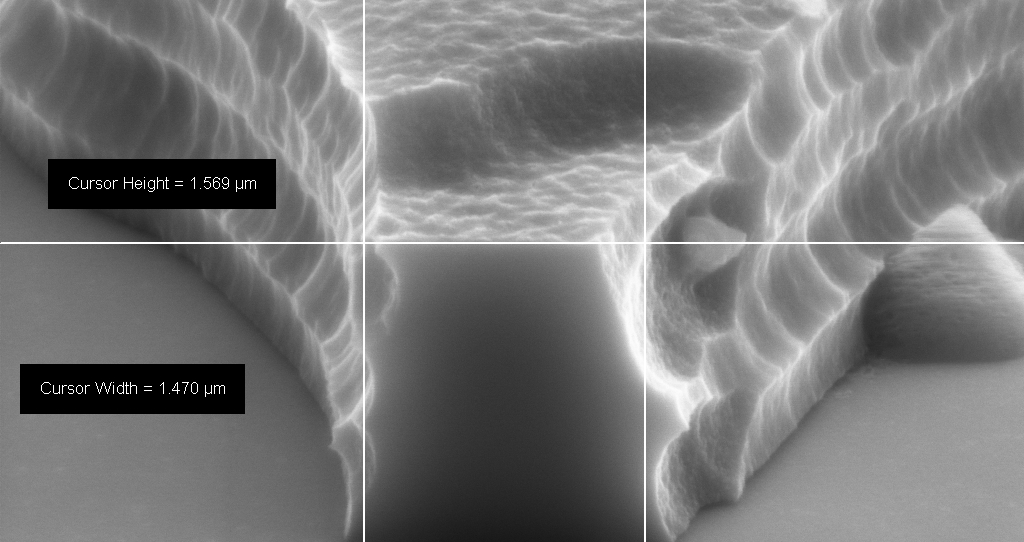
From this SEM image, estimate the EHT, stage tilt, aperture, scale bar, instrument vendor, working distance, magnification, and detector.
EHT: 8 kV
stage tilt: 70°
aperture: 30 µm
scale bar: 1000 nm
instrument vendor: Zeiss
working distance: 12 mm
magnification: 70.2 K X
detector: SE2